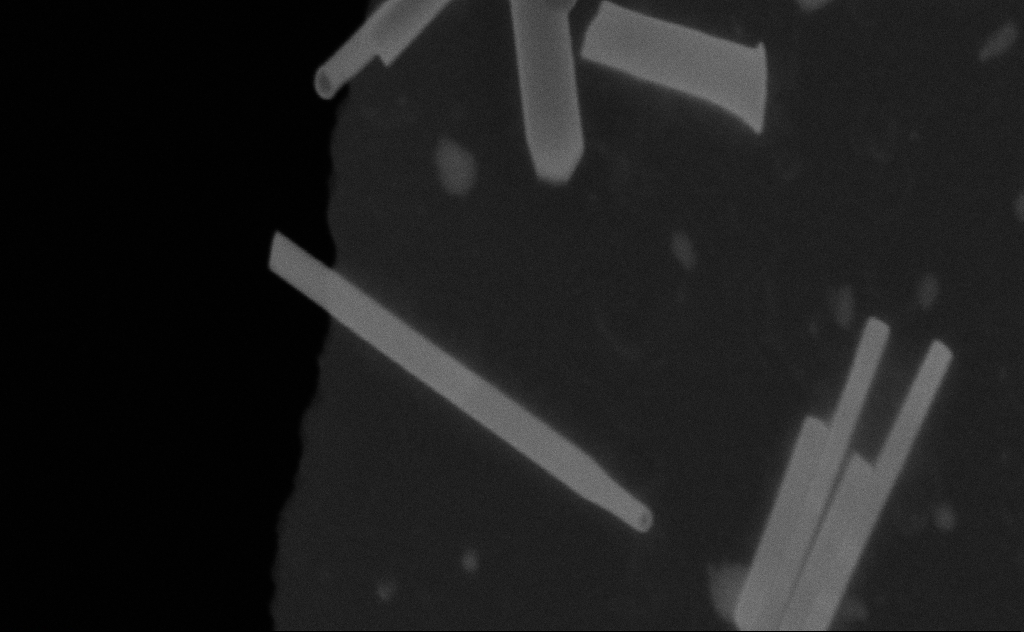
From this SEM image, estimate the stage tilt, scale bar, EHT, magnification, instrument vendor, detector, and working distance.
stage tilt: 0°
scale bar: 200 nm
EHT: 20 kV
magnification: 182.99 K X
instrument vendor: Zeiss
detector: SE2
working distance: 9 mm